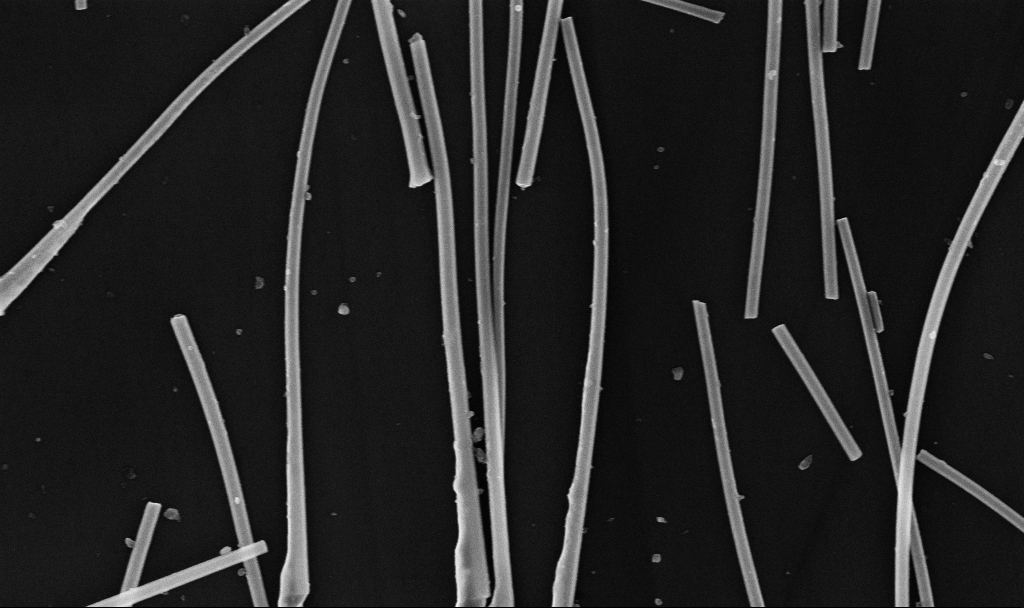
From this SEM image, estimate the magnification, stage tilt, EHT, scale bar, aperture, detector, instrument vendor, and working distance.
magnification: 47.3 K X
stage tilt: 0°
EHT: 10 kV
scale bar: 1000 nm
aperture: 30 µm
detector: InLens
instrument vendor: Zeiss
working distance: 6.7 mm